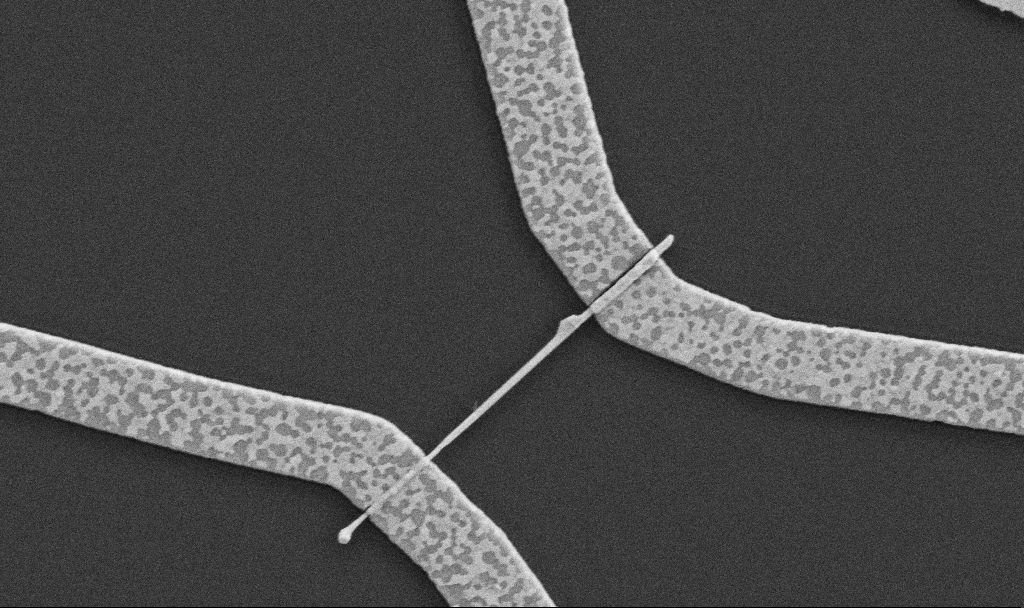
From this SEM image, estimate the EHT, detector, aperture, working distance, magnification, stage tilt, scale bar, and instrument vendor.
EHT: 5 kV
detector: SE2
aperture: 30 µm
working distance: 8.7 mm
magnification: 30 K X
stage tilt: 0°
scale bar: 1000 nm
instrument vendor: Zeiss